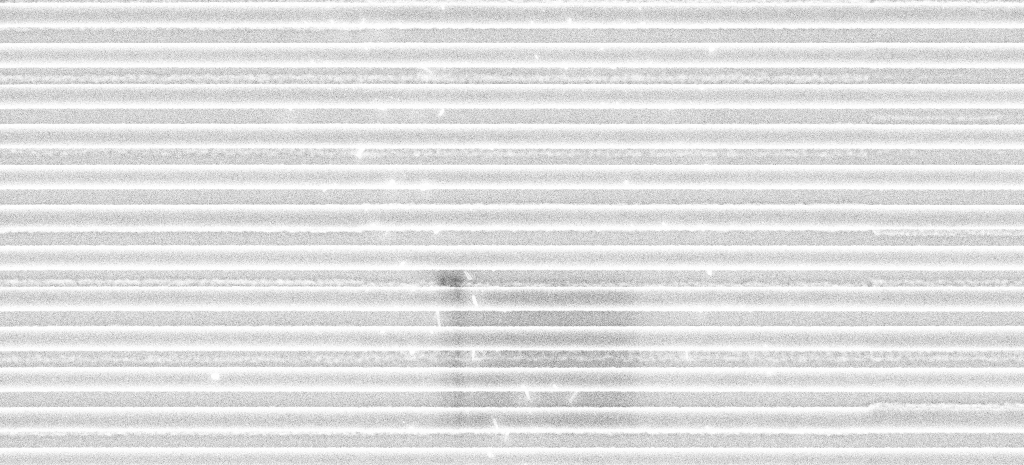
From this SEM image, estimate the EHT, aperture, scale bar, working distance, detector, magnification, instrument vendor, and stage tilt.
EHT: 5 kV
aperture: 30 µm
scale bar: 2000 nm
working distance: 10.3 mm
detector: InLens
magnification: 22.75 K X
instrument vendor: Zeiss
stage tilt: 0°